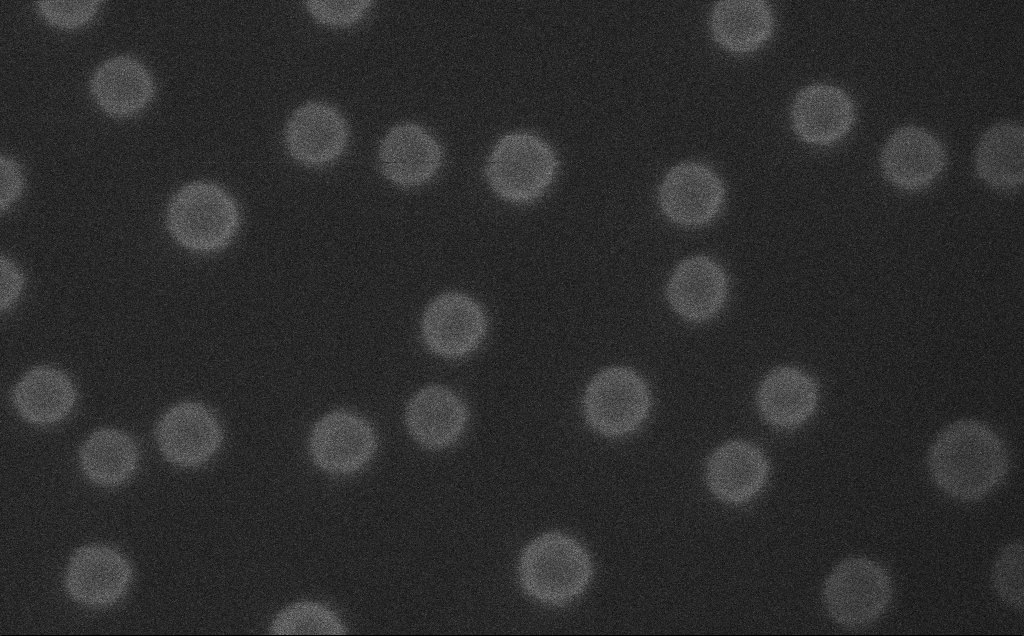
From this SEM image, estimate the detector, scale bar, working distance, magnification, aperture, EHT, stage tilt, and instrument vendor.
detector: InLens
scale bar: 20 nm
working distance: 4 mm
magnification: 800 K X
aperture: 30 µm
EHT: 10 kV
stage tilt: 0°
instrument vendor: Zeiss